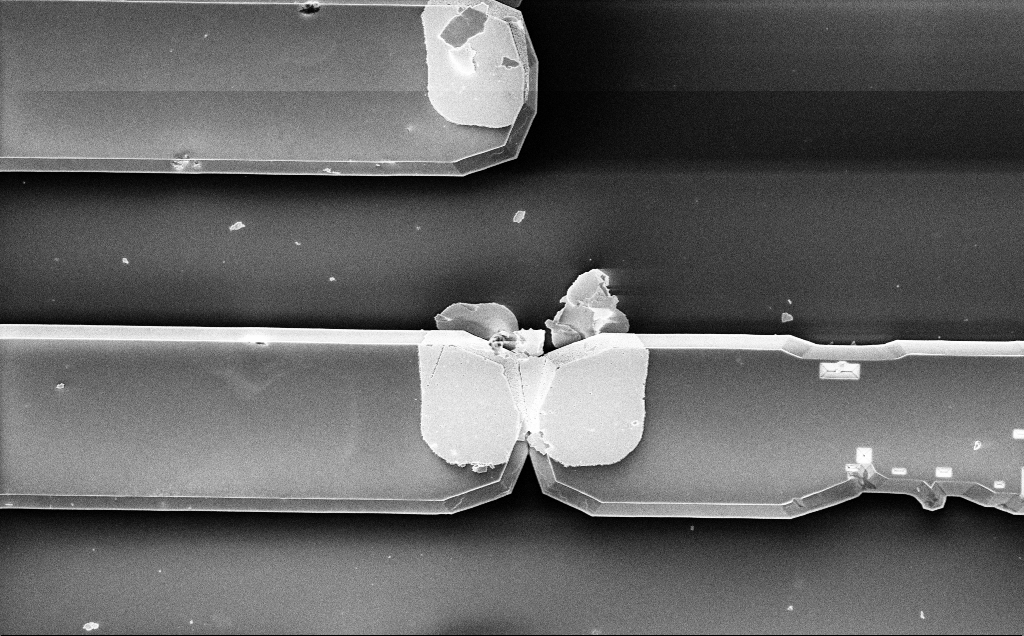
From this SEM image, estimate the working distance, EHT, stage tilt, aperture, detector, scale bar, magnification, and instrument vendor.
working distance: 15 mm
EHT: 5 kV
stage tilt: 0.2°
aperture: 30 µm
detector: InLens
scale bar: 20000 nm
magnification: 3.13 K X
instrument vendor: Zeiss